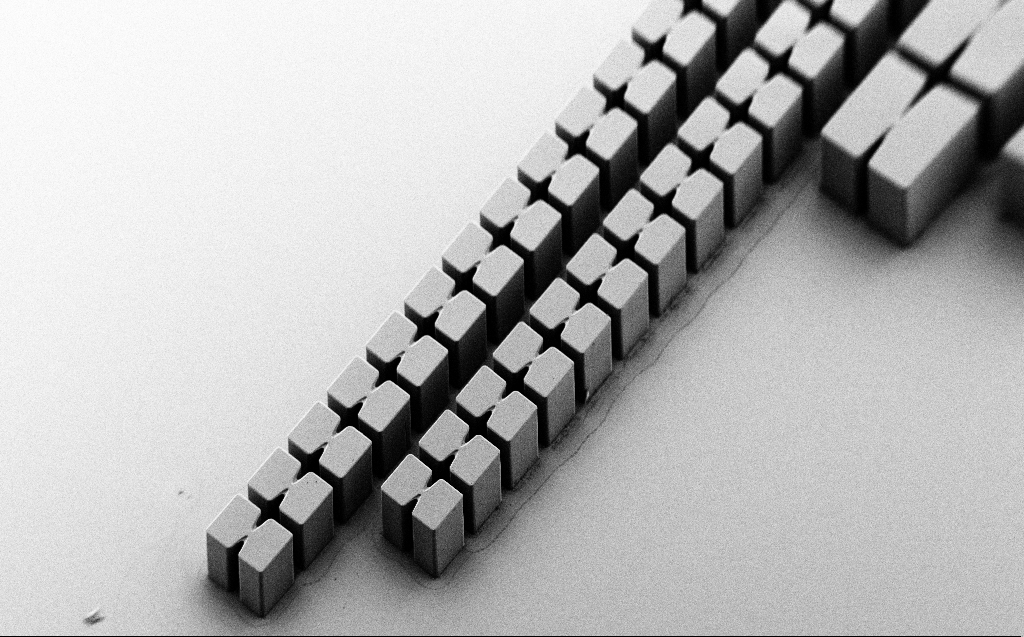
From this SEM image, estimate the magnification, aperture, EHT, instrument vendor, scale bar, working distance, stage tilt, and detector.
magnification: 0.105 K X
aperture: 30 µm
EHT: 3 kV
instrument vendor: Zeiss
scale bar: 200000 nm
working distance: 7 mm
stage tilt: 45°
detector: SE2